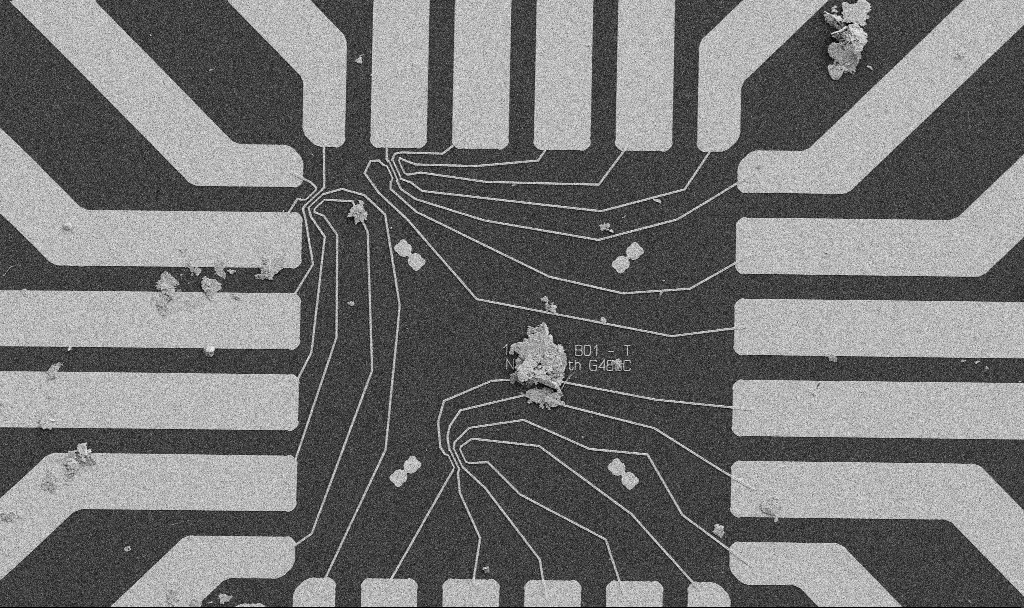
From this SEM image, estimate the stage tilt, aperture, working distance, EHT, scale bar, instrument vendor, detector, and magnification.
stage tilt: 0°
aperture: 30 µm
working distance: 10.6 mm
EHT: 5 kV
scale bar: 20000 nm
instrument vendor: Zeiss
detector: SE2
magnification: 1 K X